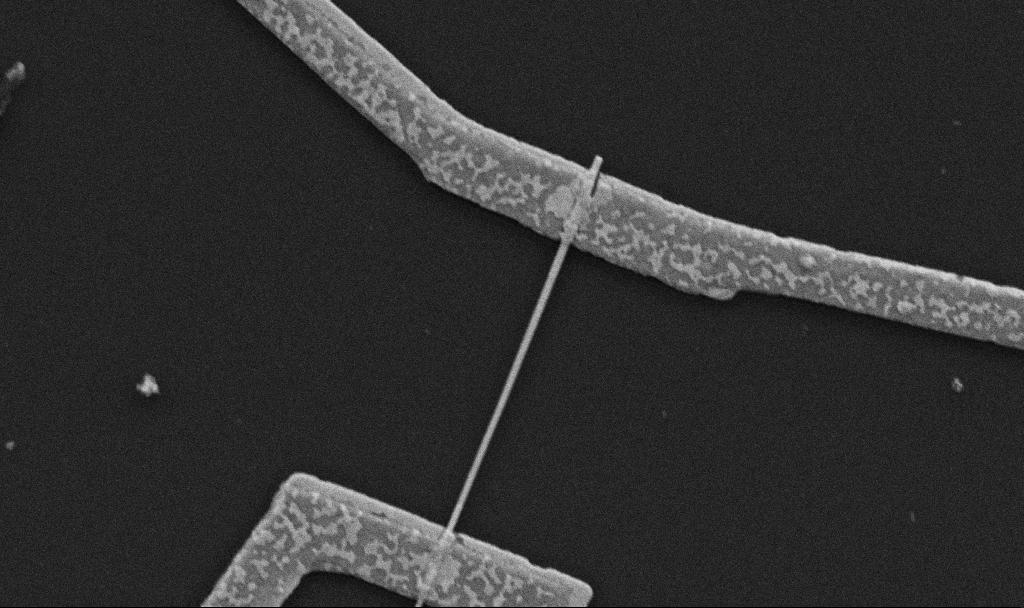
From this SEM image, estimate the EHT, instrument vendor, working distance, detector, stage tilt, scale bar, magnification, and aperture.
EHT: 5 kV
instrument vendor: Zeiss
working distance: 10.7 mm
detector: SE2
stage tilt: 0°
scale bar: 1000 nm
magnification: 30 K X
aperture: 30 µm